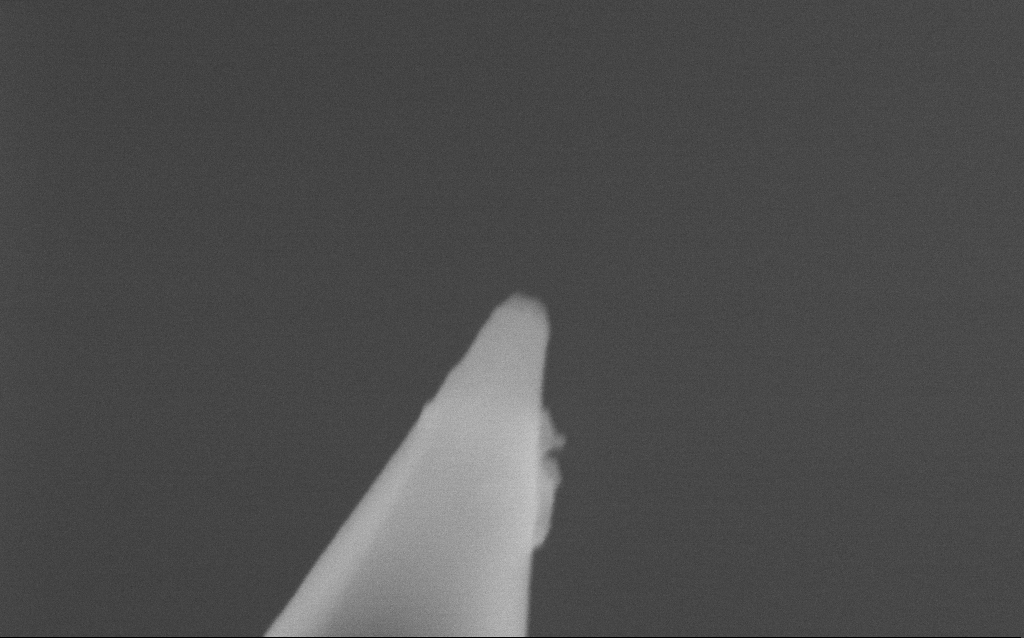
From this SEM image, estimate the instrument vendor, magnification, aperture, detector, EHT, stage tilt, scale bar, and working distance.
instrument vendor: Zeiss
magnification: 250 K X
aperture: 30 µm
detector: InLens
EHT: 5 kV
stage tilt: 0°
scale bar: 200 nm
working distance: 5.2 mm